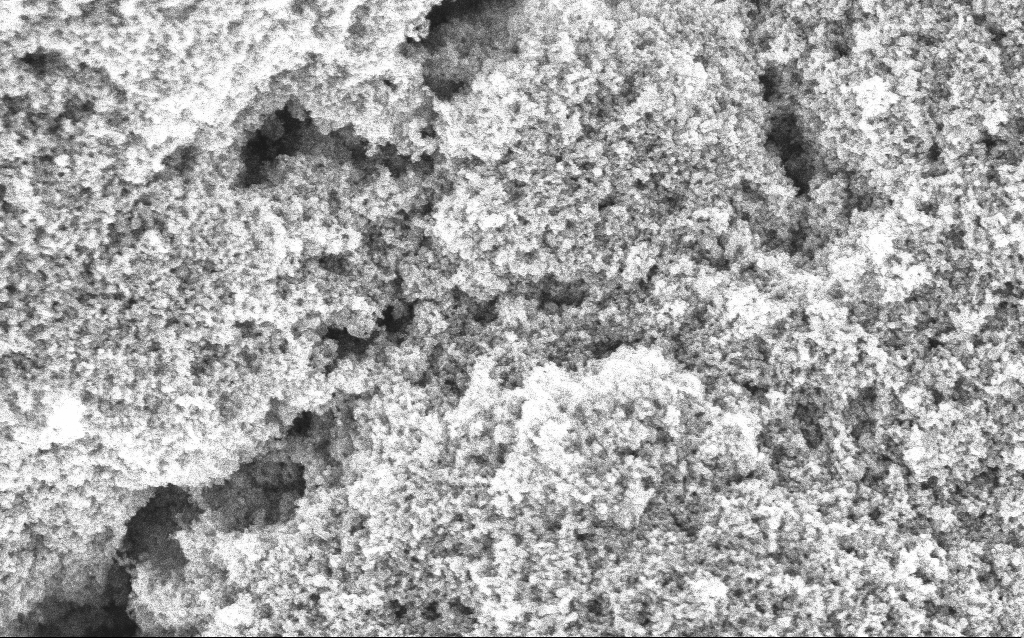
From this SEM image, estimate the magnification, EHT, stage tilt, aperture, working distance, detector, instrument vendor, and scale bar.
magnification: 65.04 K X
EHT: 5 kV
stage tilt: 0°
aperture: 30 µm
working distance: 4.2 mm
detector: InLens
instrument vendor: Zeiss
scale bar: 1000 nm